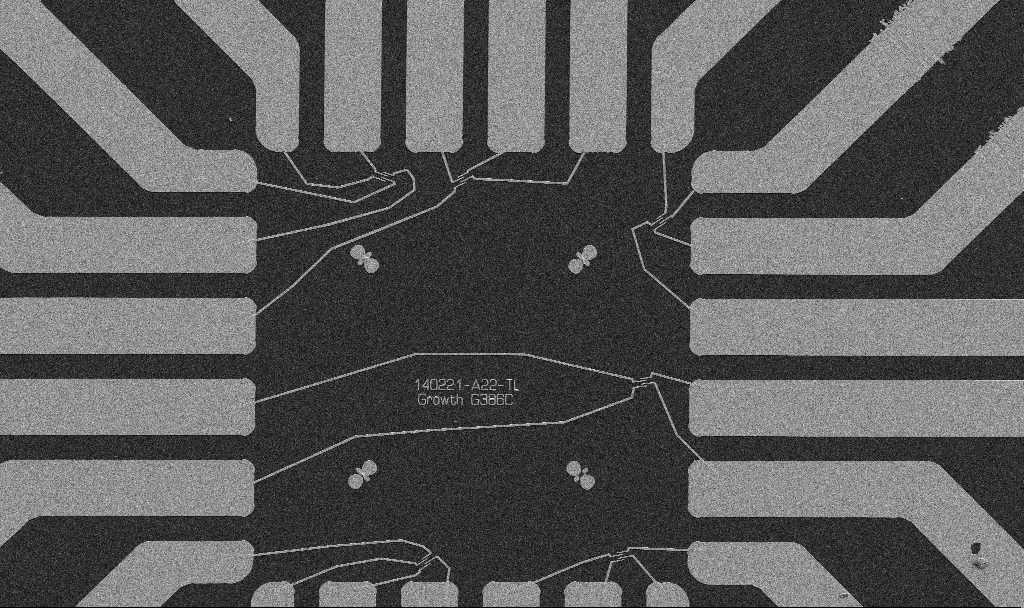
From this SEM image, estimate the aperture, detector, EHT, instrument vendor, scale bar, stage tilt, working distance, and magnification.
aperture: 30 µm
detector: SE2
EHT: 5 kV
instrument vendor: Zeiss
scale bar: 20000 nm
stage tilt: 0°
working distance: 10.7 mm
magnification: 1 K X